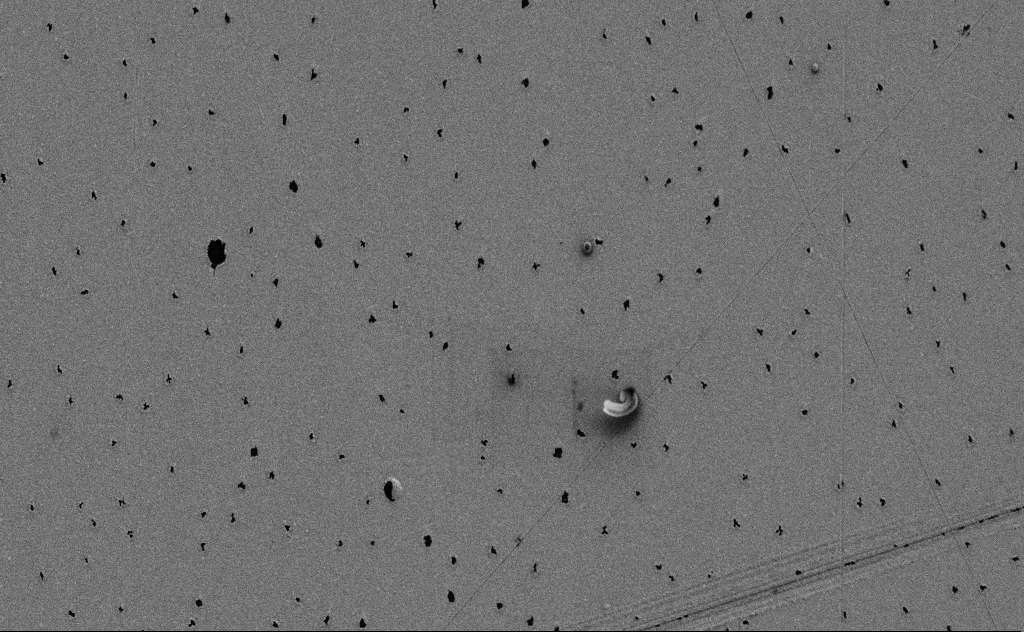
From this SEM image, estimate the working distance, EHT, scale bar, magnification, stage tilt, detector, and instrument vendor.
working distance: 10 mm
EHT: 3 kV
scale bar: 2000 nm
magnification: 7.95 K X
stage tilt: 0°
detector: SE2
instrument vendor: Zeiss